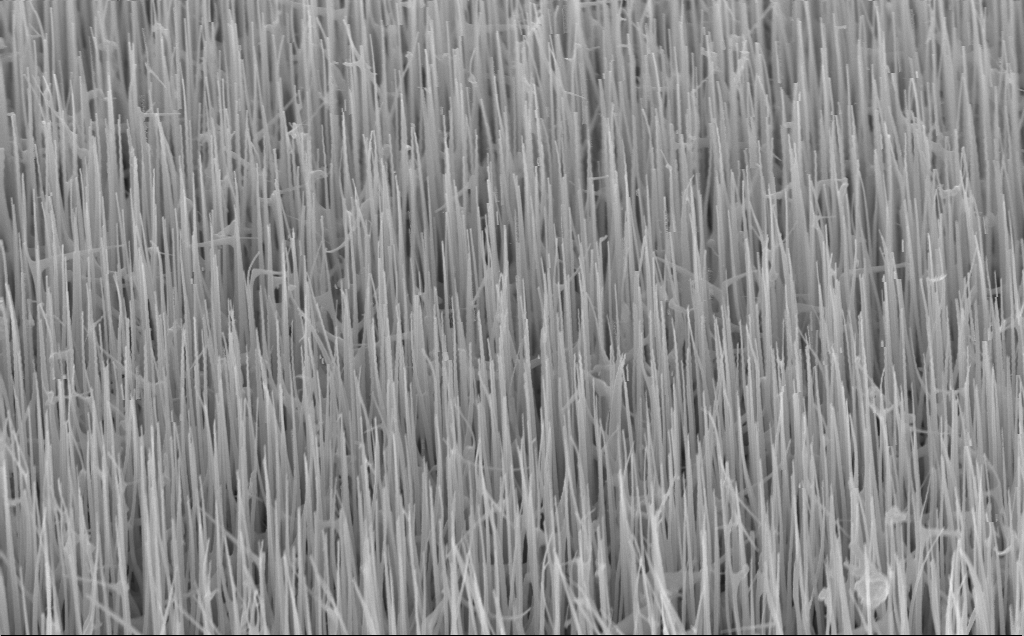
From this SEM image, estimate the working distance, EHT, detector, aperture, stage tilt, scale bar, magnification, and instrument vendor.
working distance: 7 mm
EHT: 10 kV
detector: InLens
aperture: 30 µm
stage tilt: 45°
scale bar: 1000 nm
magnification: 30.87 K X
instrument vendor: Zeiss